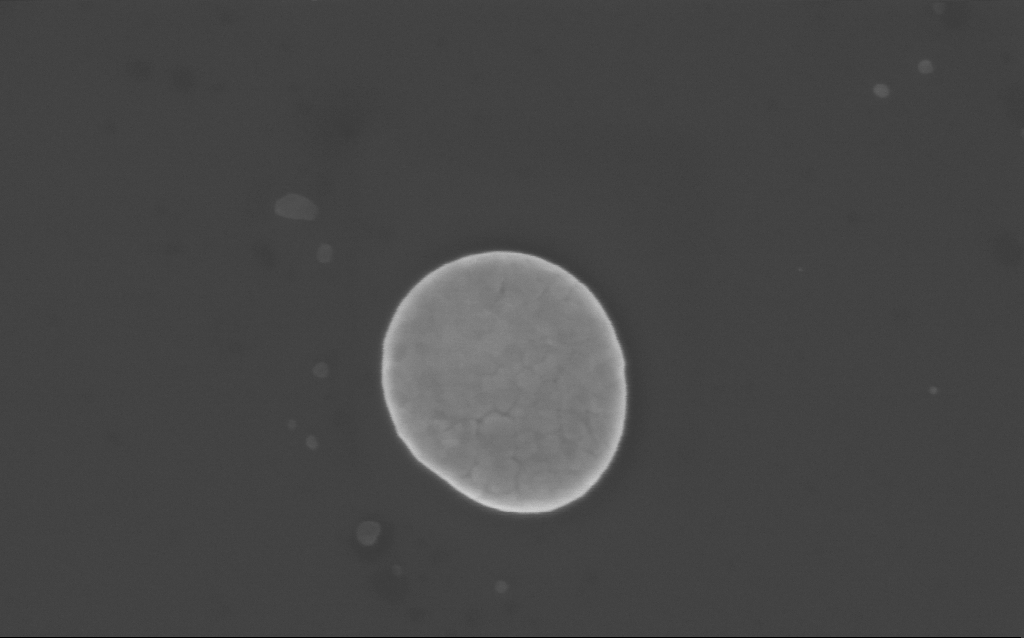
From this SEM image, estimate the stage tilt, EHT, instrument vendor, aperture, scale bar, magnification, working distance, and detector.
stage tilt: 0°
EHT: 3 kV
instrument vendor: Zeiss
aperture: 30 µm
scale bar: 200 nm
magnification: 130.4 K X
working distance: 4 mm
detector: InLens